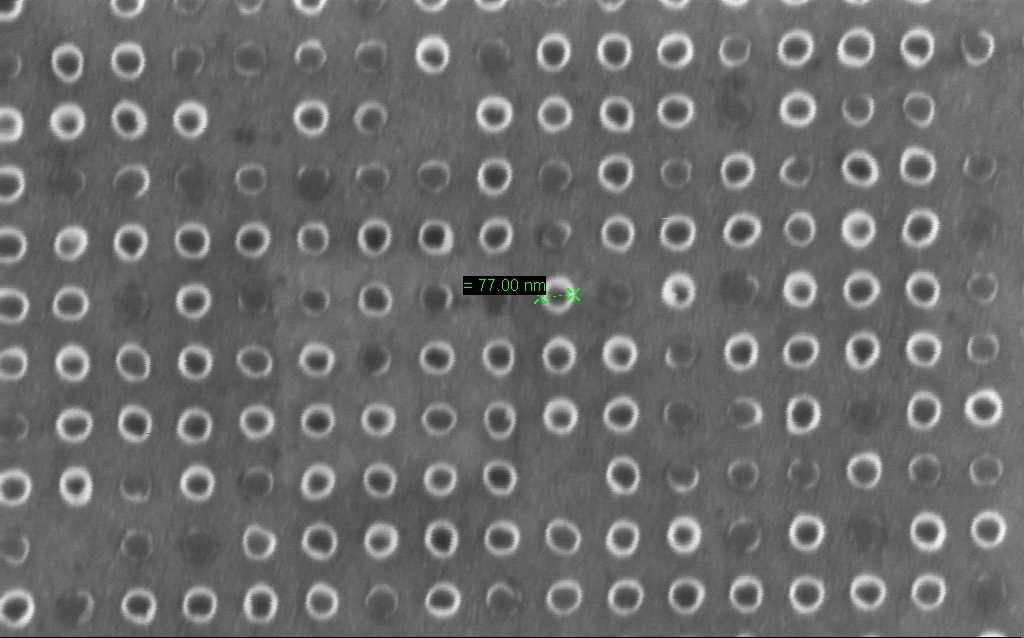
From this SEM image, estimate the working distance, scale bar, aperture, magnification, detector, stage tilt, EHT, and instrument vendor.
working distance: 5.9 mm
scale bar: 200 nm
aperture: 30 µm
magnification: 158.02 K X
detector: InLens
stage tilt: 0°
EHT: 1.5 kV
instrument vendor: Zeiss